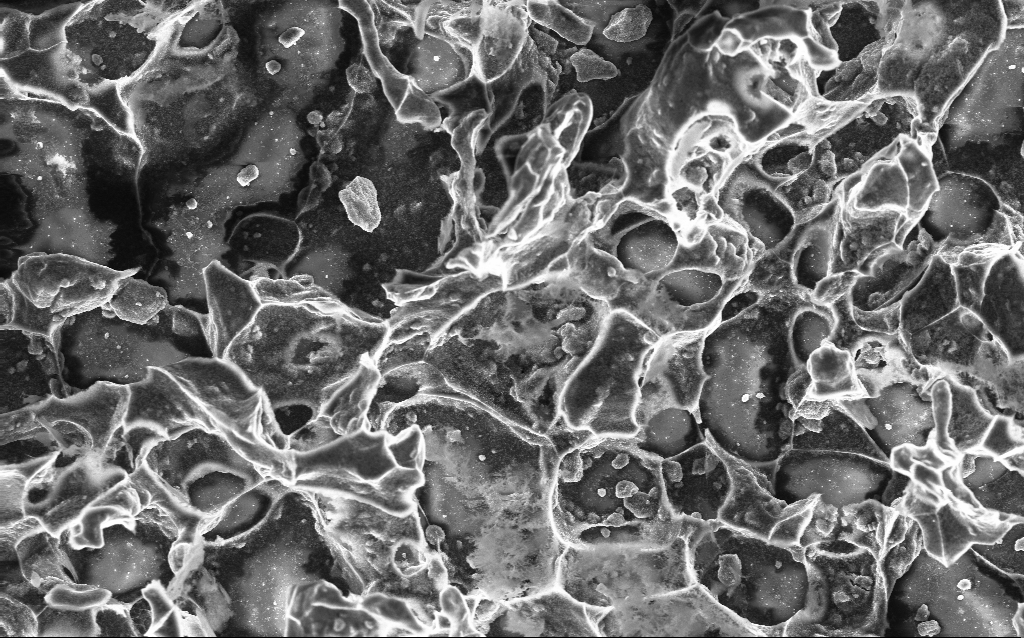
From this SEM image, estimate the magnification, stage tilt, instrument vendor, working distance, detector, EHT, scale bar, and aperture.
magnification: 1.96 K X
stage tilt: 0°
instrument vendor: Zeiss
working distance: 2.8 mm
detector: InLens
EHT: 10 kV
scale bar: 10000 nm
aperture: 30 µm